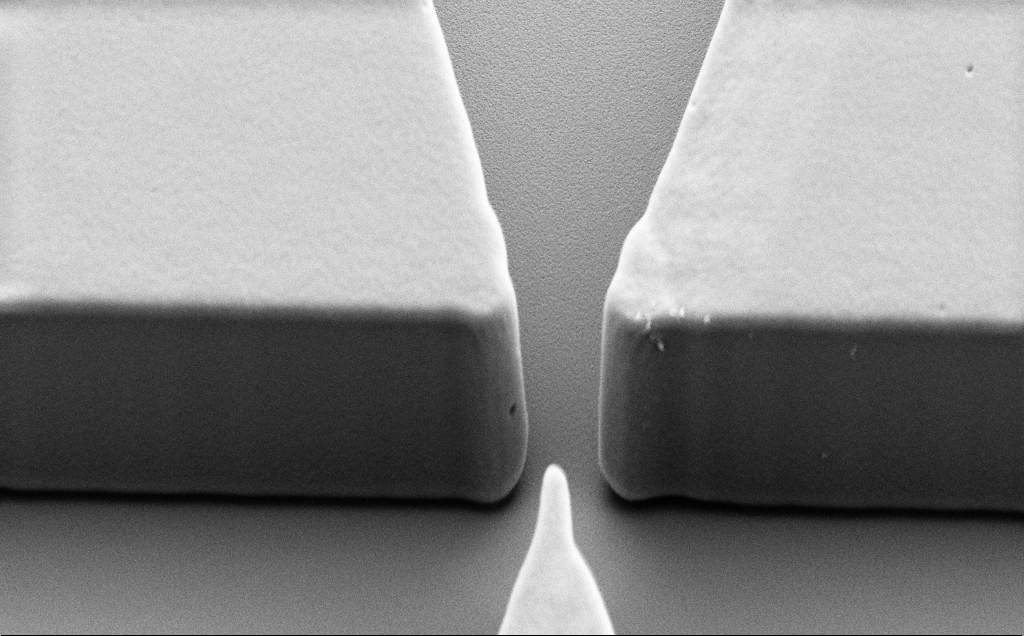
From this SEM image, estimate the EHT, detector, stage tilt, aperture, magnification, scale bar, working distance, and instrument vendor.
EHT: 5 kV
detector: SE2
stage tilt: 40°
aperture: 30 µm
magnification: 11.47 K X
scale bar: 2000 nm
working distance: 9 mm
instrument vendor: Zeiss